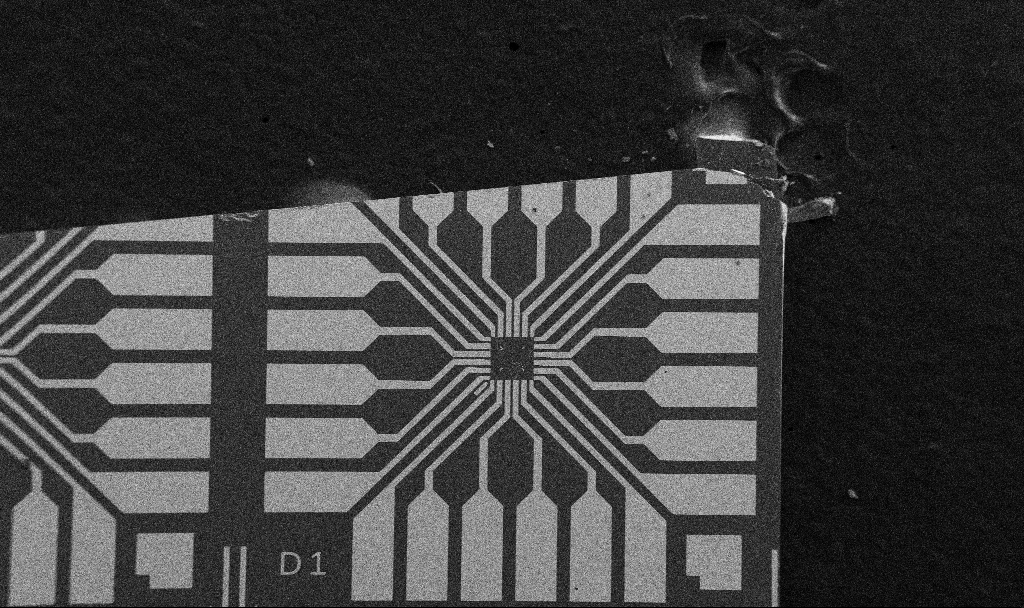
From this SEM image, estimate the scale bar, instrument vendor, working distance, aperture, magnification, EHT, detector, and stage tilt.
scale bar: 200000 nm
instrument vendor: Zeiss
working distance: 10.7 mm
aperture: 30 µm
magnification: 0.1 K X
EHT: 5 kV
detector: SE2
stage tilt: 0°